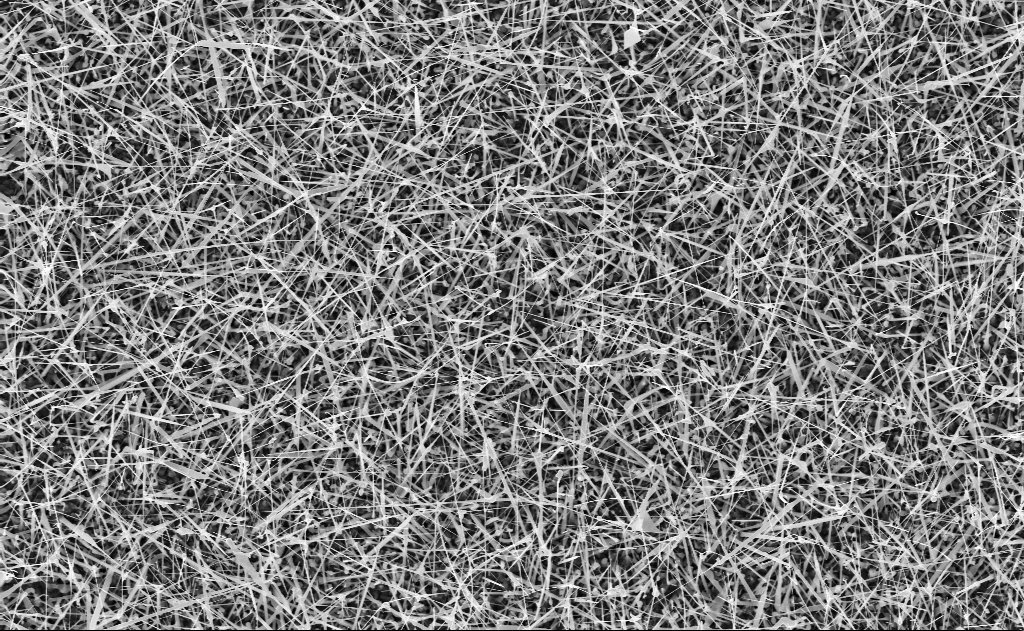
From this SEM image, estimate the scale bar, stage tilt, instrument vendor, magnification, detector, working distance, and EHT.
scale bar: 2000 nm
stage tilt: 0°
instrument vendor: Zeiss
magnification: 10 K X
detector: InLens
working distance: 10 mm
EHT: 10 kV